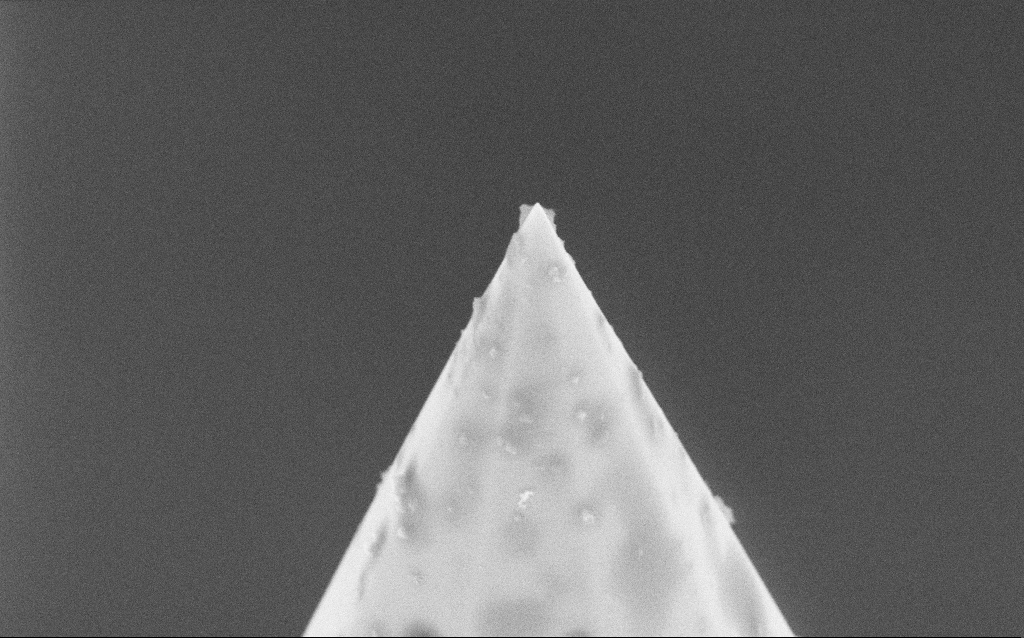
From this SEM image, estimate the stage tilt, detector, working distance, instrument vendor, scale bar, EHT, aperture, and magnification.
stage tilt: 45°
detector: InLens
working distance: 11.8 mm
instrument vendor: Zeiss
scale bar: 2000 nm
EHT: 5 kV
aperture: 30 µm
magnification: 27.33 K X